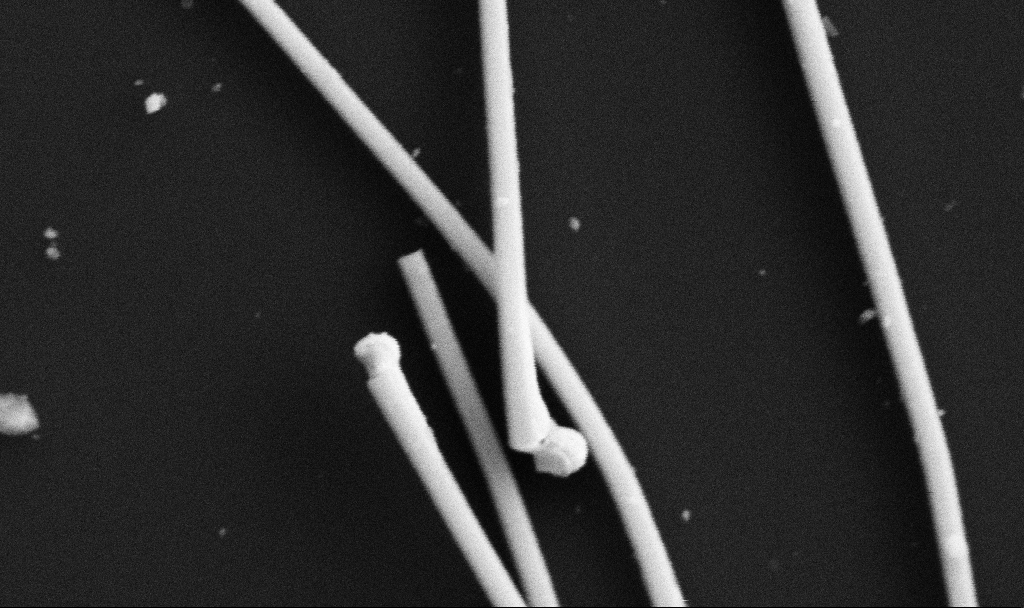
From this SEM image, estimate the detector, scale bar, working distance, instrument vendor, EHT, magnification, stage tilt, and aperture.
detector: SE2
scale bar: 200 nm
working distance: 10.7 mm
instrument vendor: Zeiss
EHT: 5 kV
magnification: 93.7 K X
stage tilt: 0°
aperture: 30 µm